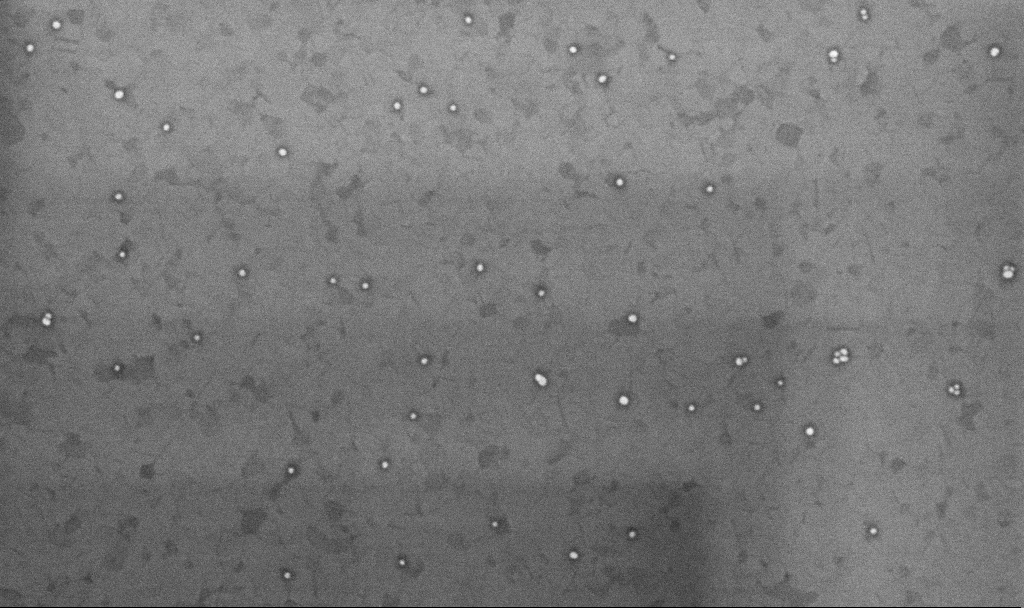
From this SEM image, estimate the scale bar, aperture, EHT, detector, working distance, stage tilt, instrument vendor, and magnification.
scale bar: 200 nm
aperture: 30 µm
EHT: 10 kV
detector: InLens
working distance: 3.3 mm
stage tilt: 0°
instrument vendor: Zeiss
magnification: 100.38 K X